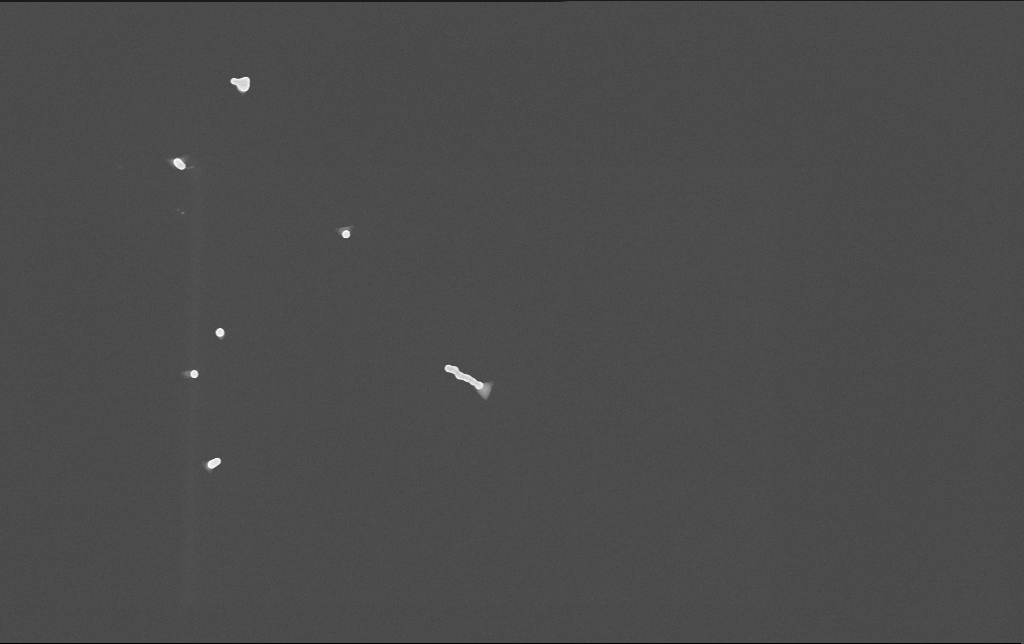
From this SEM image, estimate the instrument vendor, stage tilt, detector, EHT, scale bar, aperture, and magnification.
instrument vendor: Zeiss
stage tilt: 0°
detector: InLens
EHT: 10 kV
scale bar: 1000 nm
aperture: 30 µm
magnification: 50 K X